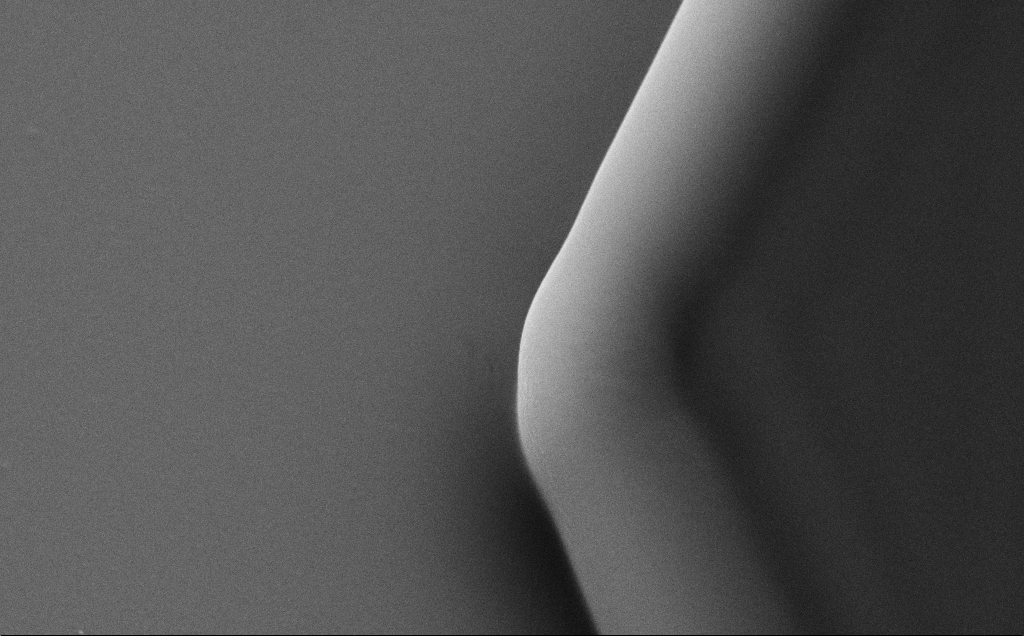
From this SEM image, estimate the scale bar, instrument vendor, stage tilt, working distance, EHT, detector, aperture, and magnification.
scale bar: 1000 nm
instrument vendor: Zeiss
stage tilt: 35°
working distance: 8 mm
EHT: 10 kV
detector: SE2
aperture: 30 µm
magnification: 36.69 K X